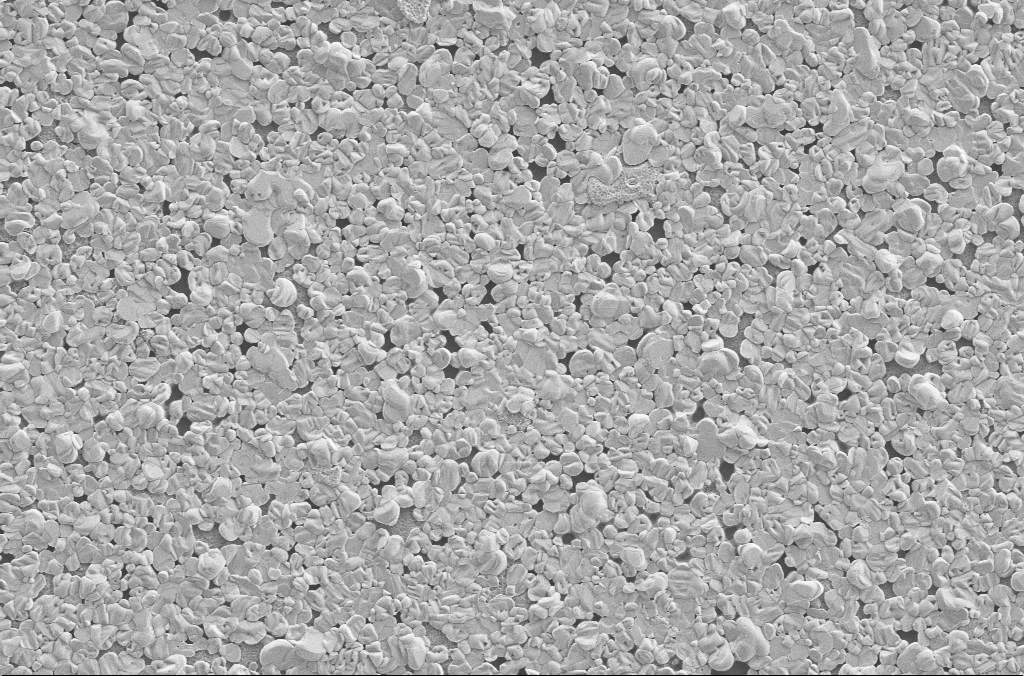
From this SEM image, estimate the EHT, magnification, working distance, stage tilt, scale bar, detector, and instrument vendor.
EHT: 2 kV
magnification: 10 K X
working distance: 3 mm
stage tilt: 0°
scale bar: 2000 nm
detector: SE2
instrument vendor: Zeiss